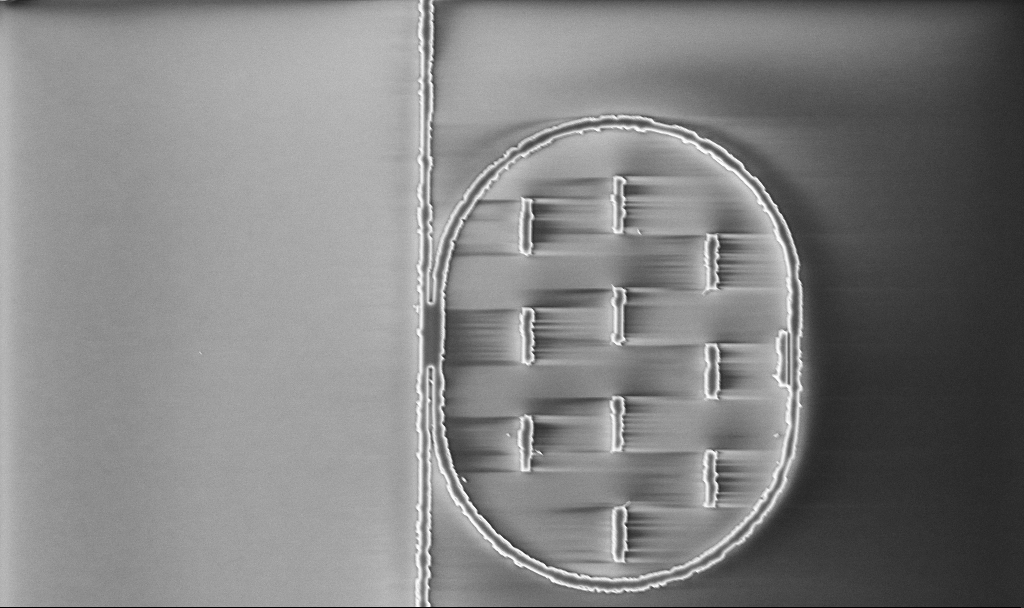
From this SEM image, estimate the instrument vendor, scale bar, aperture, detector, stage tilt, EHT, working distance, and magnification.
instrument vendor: Zeiss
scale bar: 10000 nm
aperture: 30 µm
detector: InLens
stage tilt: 0°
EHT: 5 kV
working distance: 10.1 mm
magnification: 6.77 K X